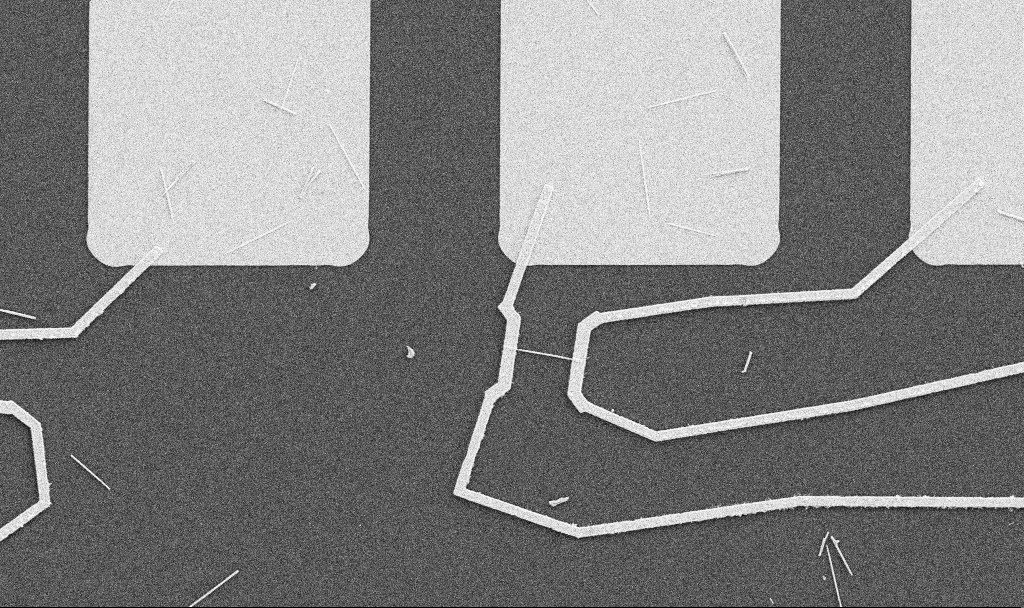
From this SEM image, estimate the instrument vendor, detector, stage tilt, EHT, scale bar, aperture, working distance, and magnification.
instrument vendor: Zeiss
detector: SE2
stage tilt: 0°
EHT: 5 kV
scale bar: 10000 nm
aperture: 30 µm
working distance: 10.7 mm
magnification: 5 K X